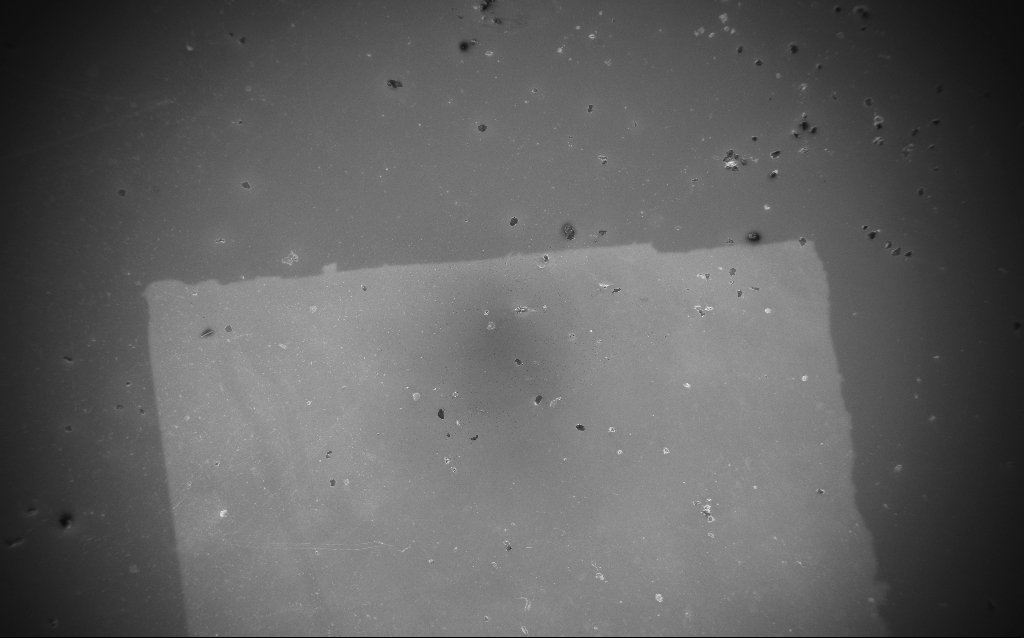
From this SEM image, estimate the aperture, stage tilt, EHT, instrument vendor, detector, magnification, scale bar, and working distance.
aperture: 30 µm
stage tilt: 0°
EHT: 5 kV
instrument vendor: Zeiss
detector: InLens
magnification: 0.096 K X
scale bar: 200000 nm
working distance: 5 mm